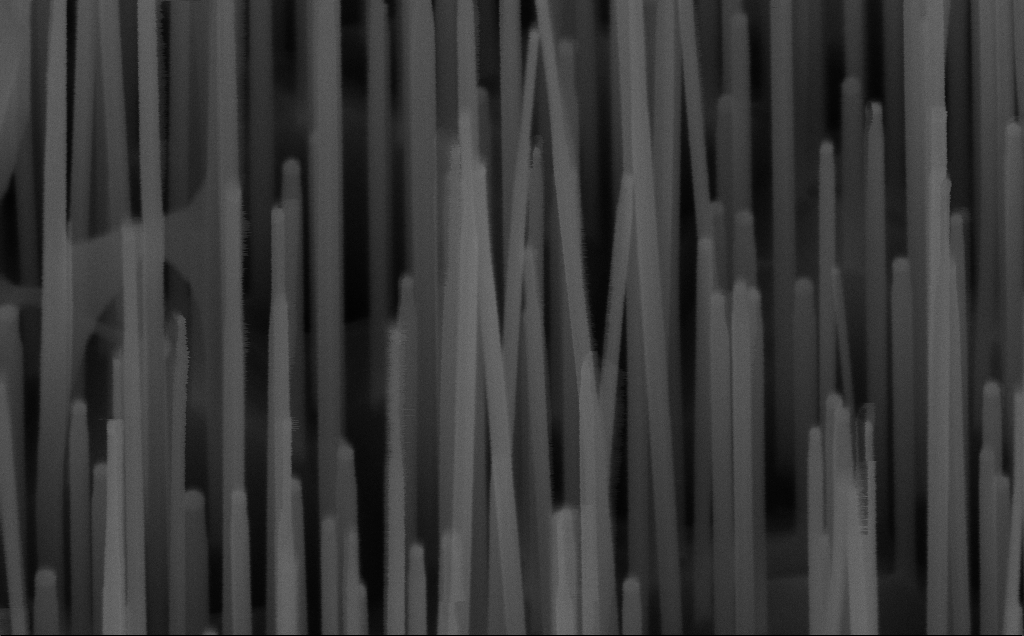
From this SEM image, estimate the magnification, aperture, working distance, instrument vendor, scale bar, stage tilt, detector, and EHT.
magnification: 200 K X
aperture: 30 µm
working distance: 7 mm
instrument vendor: Zeiss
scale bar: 200 nm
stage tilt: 45°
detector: InLens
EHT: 10 kV